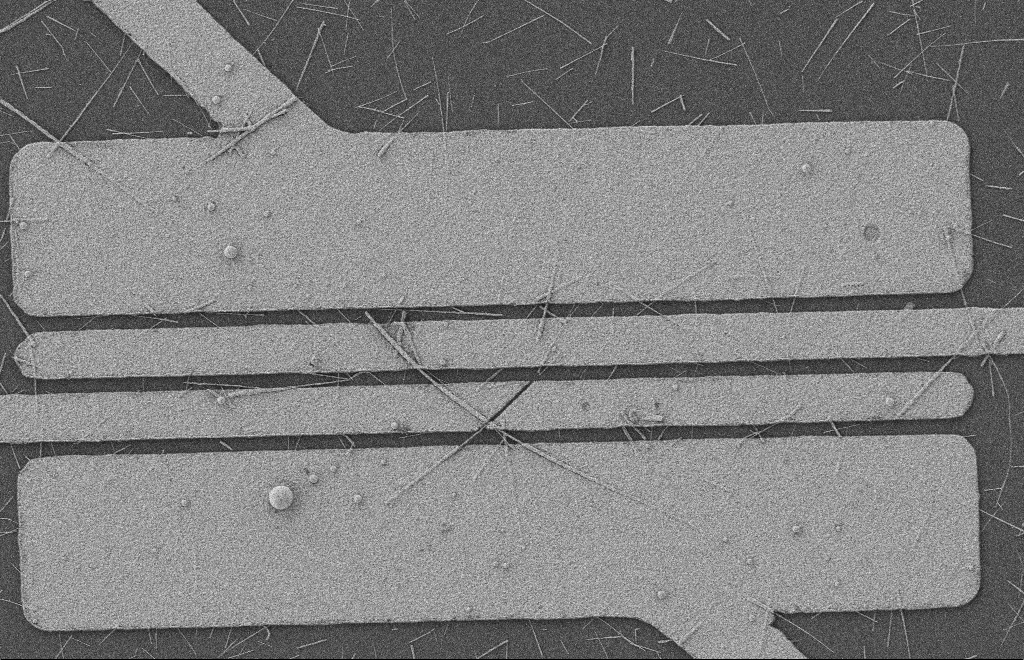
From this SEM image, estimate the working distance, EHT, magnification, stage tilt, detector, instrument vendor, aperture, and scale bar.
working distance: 8 mm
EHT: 2 kV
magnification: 5.73 K X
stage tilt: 0°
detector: SE2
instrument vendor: Zeiss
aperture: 20 µm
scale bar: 2000 nm